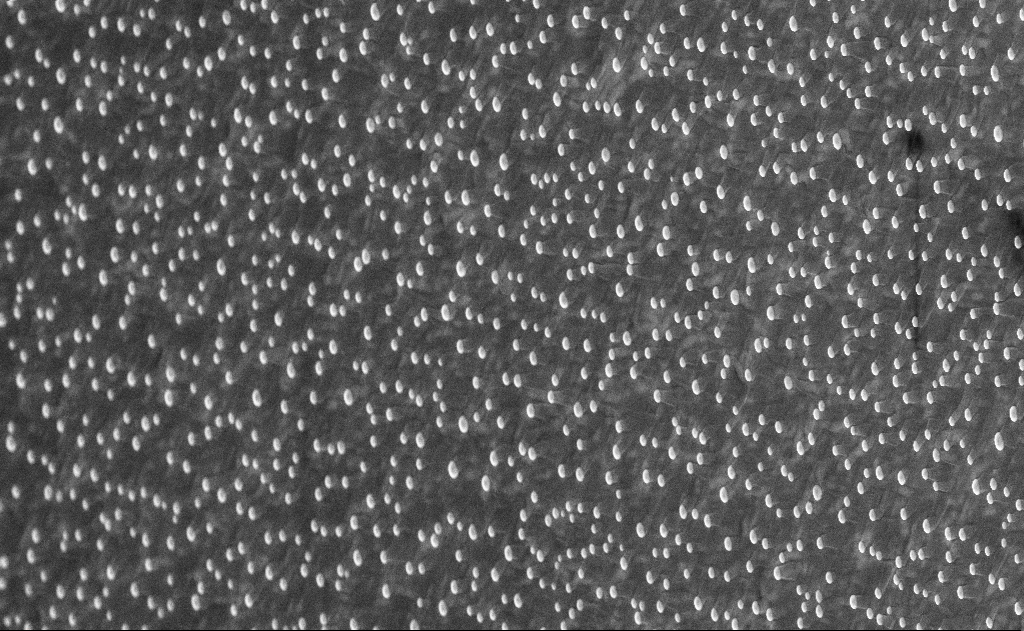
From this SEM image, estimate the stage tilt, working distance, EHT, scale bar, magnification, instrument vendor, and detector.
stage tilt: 0°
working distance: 12 mm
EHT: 10 kV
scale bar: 10000 nm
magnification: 5 K X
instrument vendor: Zeiss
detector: InLens